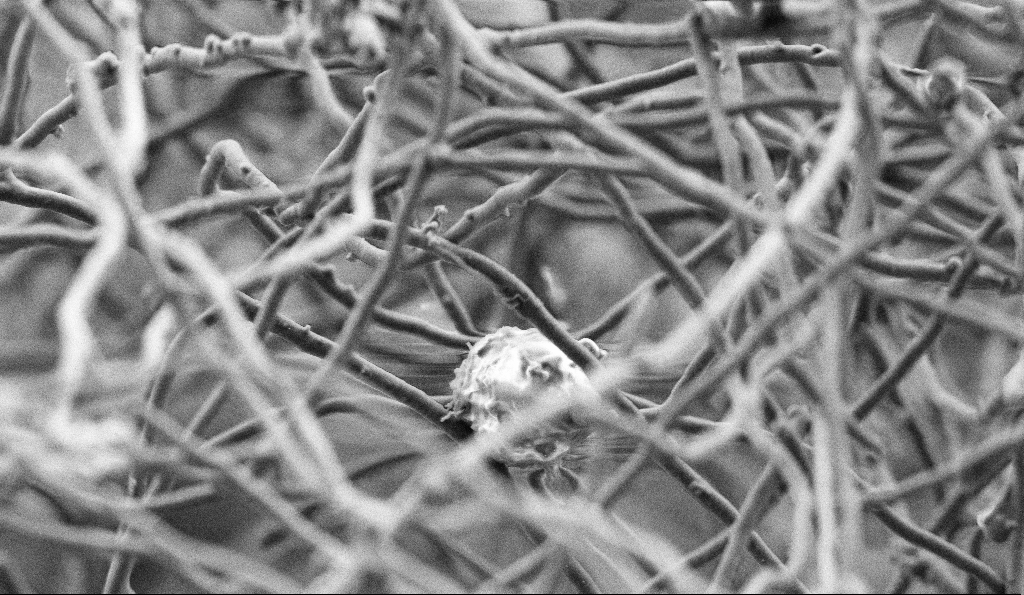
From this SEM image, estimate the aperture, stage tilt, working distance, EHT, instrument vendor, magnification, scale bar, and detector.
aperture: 30 µm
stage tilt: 0°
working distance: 5 mm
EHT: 3 kV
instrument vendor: Zeiss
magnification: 10 K X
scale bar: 2000 nm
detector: SE2